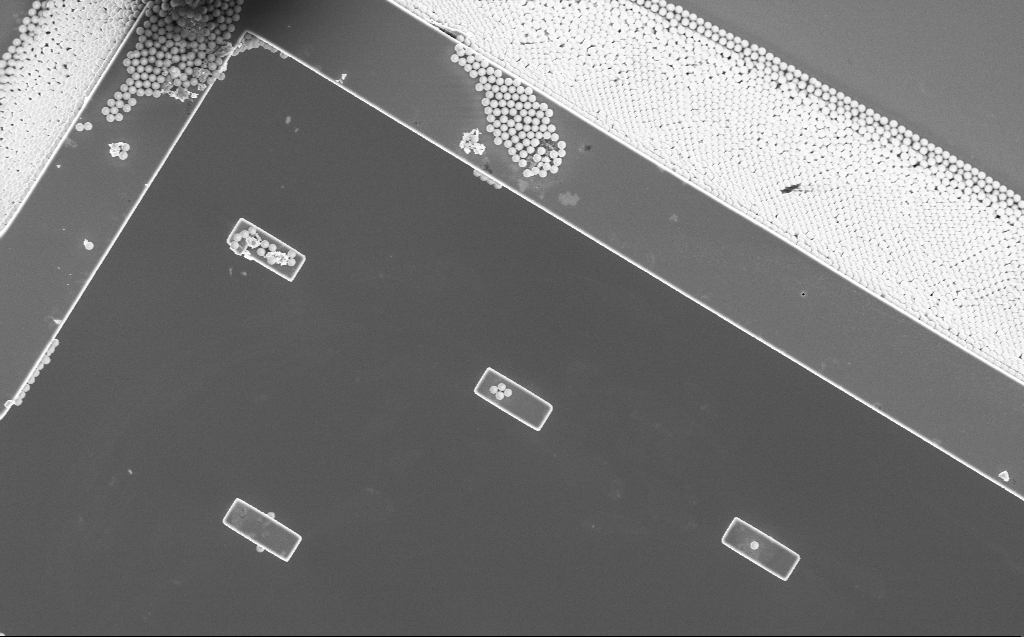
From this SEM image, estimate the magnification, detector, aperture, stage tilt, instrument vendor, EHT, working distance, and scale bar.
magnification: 3.74 K X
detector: InLens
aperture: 30 µm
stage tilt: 0°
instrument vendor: Zeiss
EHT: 5 kV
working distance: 8 mm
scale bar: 10000 nm